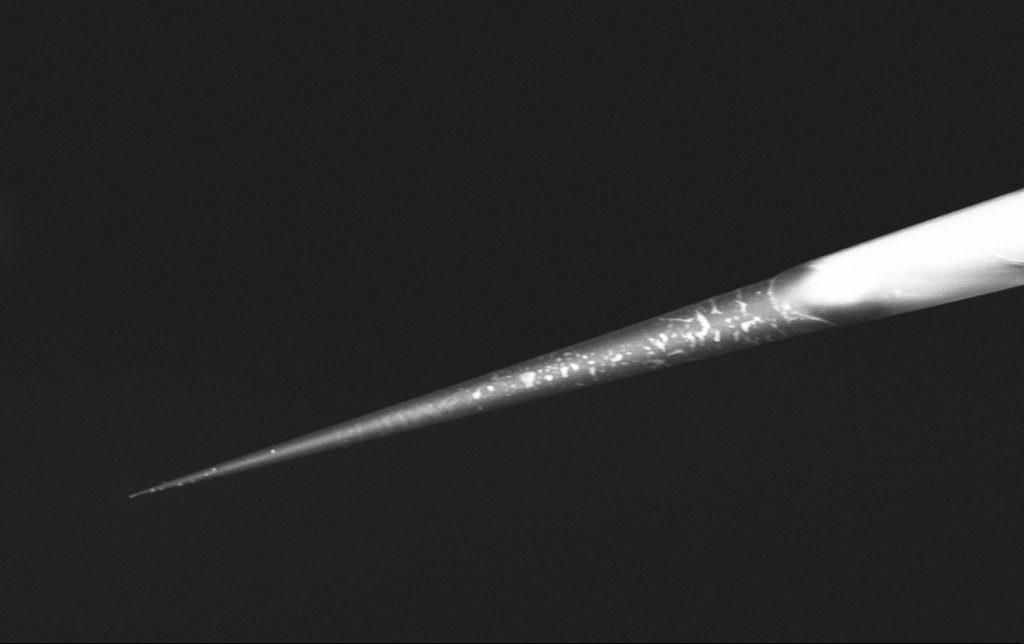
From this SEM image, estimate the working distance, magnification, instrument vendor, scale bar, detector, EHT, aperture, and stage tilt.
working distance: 6.3 mm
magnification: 5 K X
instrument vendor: Zeiss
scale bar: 10000 nm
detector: InLens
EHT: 3 kV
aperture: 30 µm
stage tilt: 0°